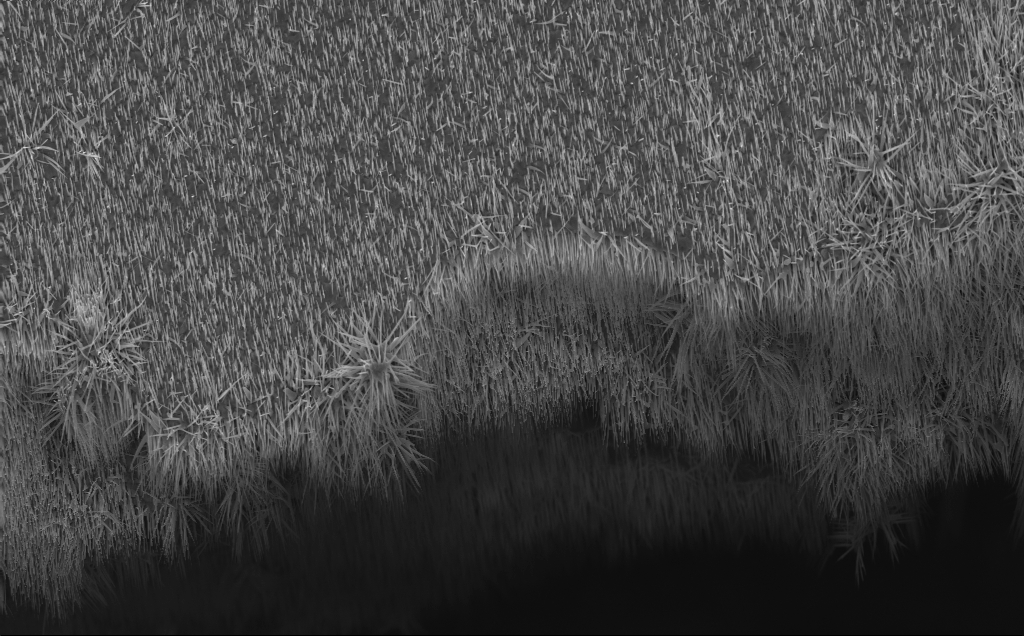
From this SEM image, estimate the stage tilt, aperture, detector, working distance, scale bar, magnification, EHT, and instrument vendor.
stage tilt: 0°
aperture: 30 µm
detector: InLens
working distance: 7 mm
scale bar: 10000 nm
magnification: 4.22 K X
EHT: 10 kV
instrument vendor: Zeiss